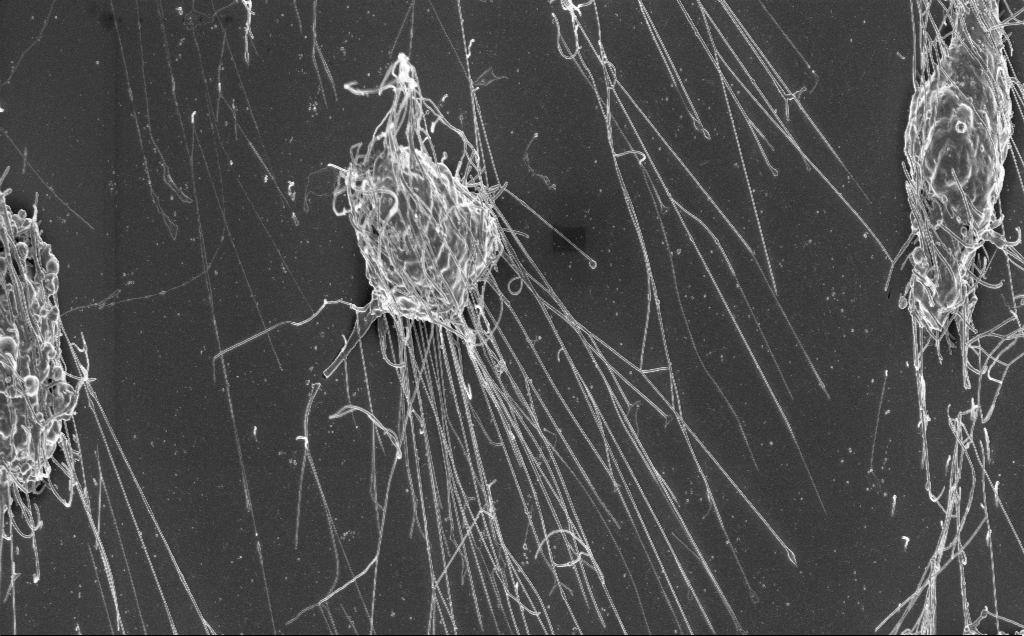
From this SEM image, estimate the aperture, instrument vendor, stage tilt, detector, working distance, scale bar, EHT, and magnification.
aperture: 30 µm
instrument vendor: Zeiss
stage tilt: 0.8°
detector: InLens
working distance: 6 mm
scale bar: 10000 nm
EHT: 3 kV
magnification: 5.22 K X